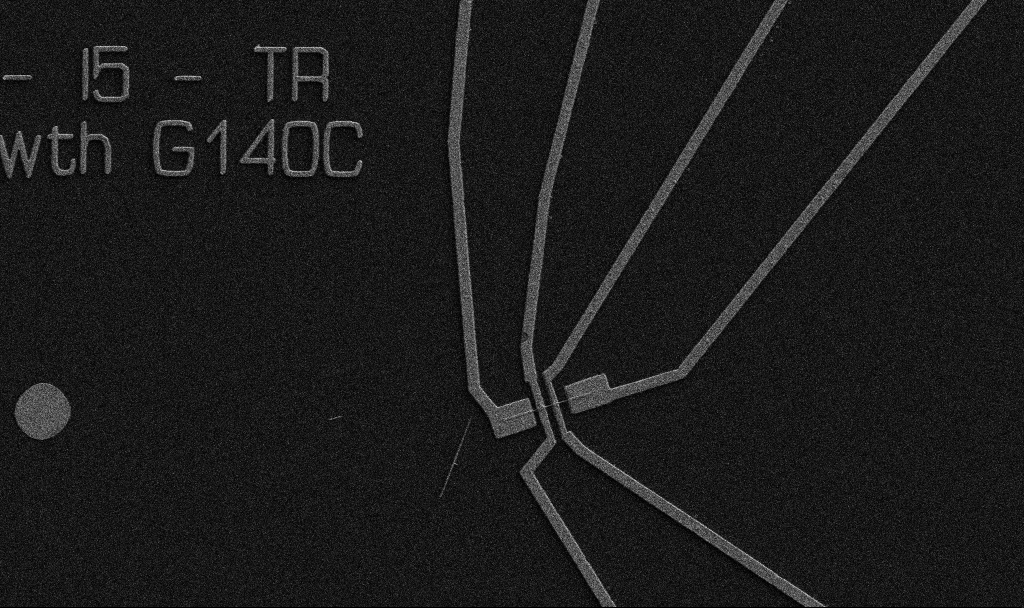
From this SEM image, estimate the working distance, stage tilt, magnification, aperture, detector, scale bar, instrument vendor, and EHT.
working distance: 10.7 mm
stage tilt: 0°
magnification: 5 K X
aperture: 30 µm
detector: SE2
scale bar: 10000 nm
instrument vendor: Zeiss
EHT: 5 kV